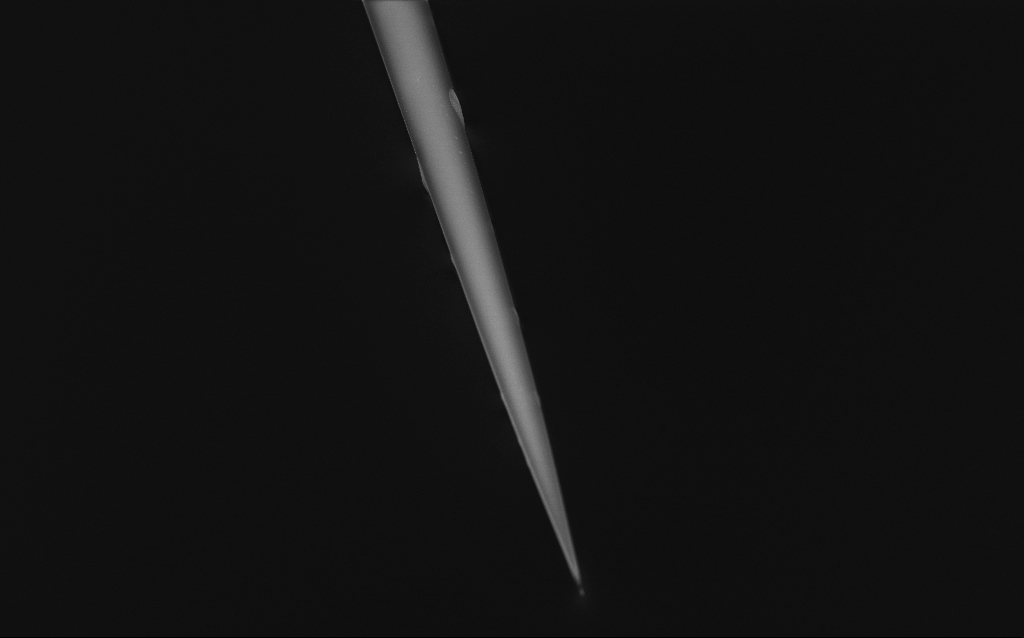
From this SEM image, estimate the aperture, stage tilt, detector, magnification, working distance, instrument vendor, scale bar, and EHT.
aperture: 30 µm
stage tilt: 45°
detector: InLens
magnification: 1 K X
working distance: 6 mm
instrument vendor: Zeiss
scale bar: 20000 nm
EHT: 1 kV